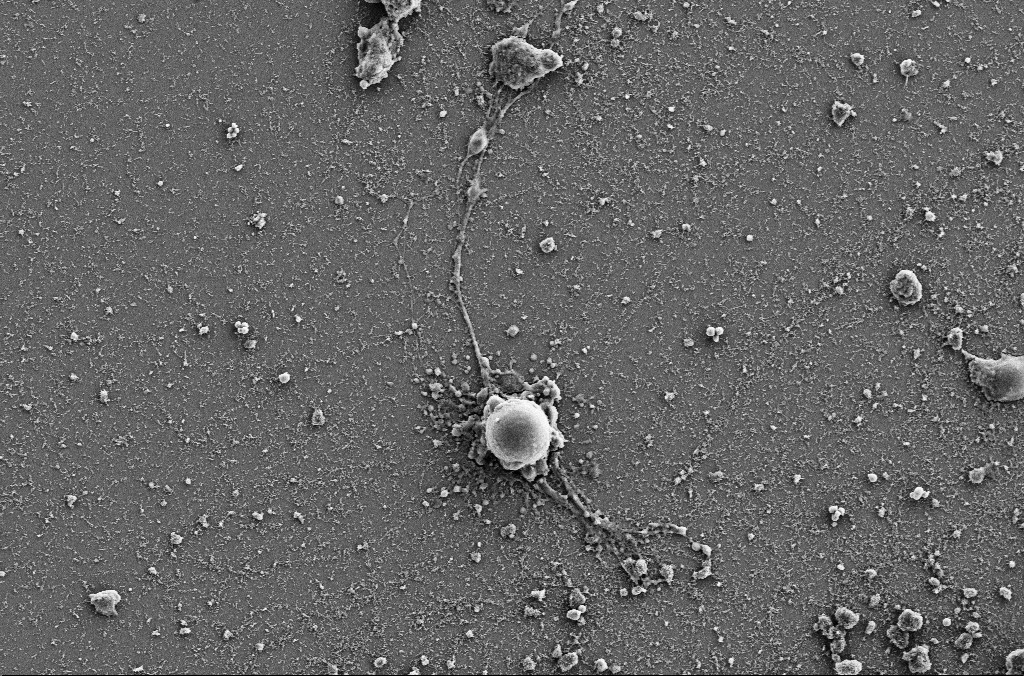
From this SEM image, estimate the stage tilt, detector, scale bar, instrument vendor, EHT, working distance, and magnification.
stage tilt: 0°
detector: SE2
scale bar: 10000 nm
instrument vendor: Zeiss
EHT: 5 kV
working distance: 4 mm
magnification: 3 K X